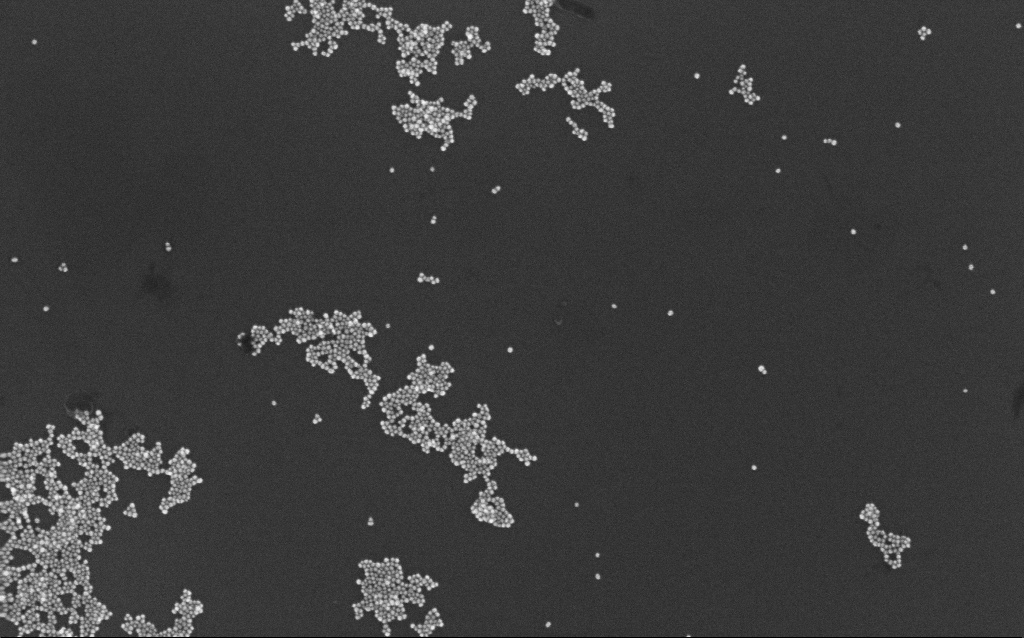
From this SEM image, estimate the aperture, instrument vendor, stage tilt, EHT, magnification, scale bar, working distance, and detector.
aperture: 30 µm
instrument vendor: Zeiss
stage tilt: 0°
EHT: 10 kV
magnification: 100 K X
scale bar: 200 nm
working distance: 7 mm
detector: InLens